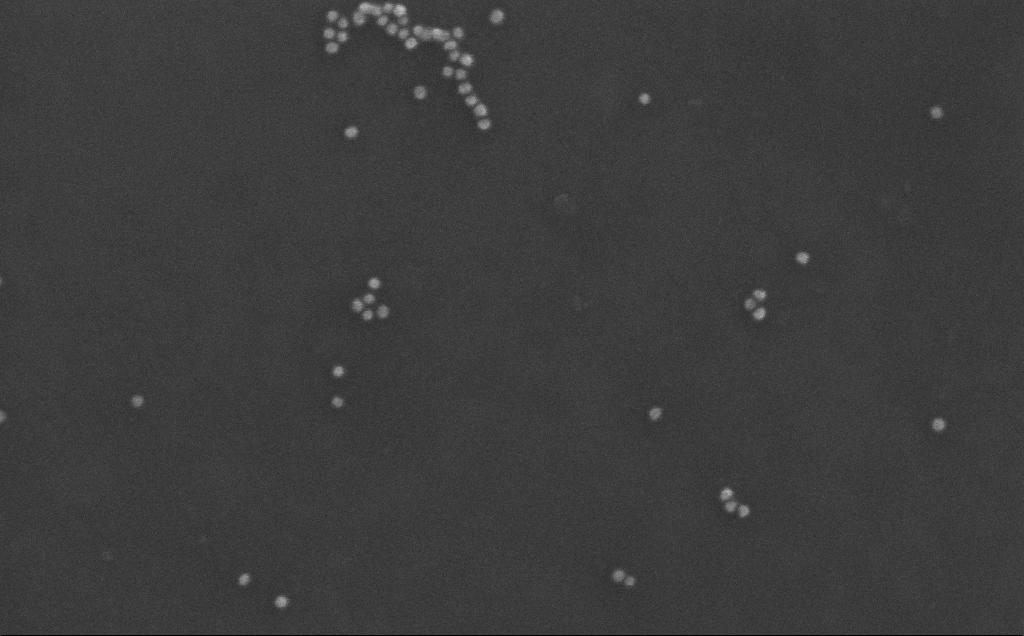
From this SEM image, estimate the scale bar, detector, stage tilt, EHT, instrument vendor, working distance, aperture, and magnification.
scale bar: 200 nm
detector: InLens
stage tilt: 0°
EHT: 10 kV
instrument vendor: Zeiss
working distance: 3 mm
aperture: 30 µm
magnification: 259.38 K X